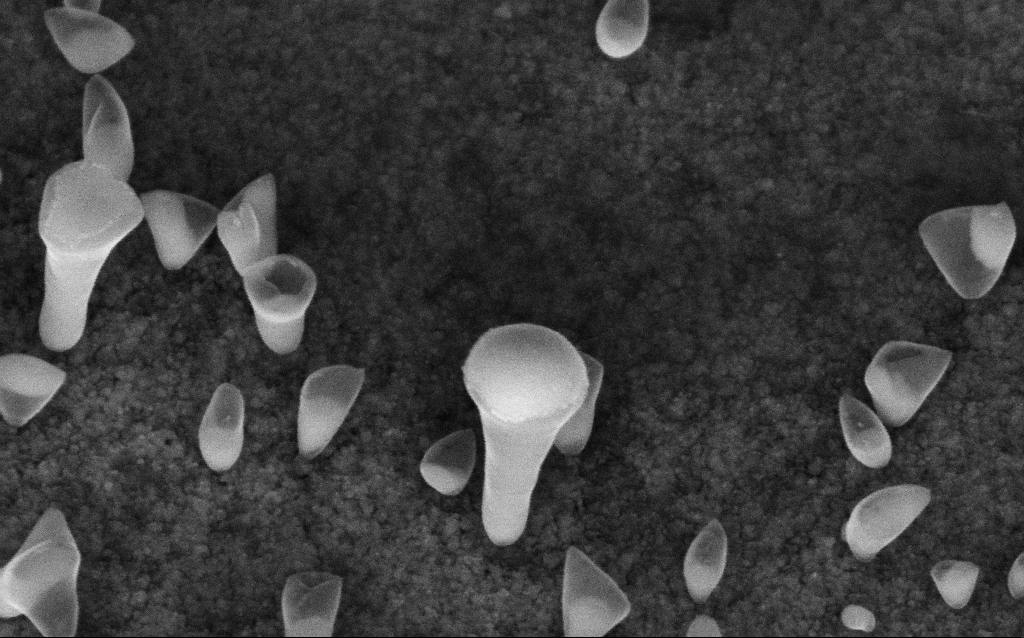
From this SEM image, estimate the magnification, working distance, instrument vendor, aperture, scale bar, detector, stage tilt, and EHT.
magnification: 161.86 K X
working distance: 7.3 mm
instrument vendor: Zeiss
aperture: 30 µm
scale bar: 100 nm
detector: InLens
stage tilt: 45°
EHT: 5 kV